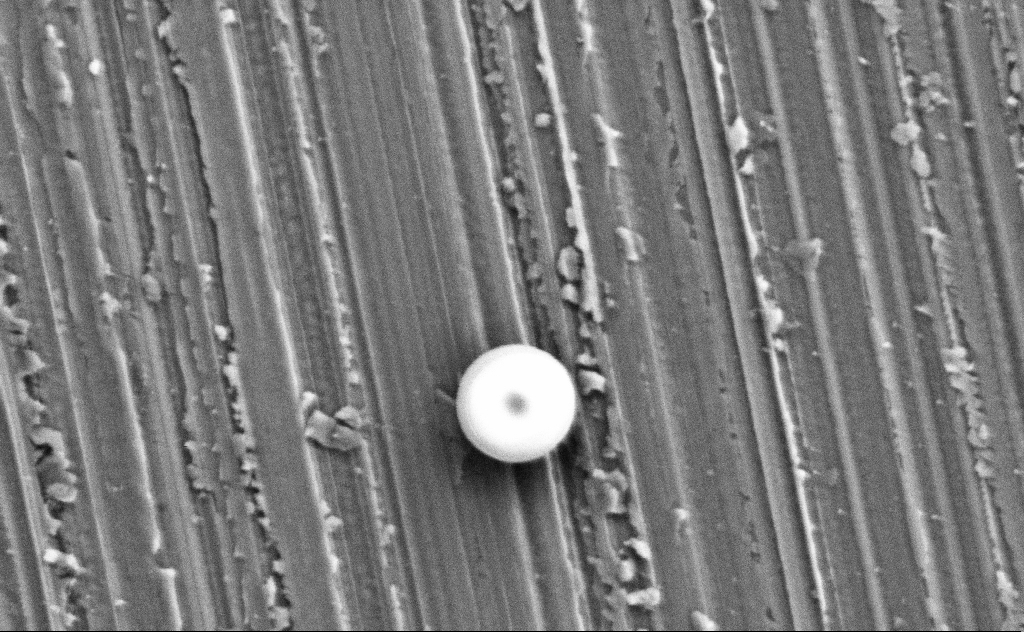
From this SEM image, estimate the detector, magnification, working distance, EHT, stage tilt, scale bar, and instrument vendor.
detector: SE2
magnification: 33.22 K X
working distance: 14 mm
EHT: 3 kV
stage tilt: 0°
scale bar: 2000 nm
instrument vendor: Zeiss